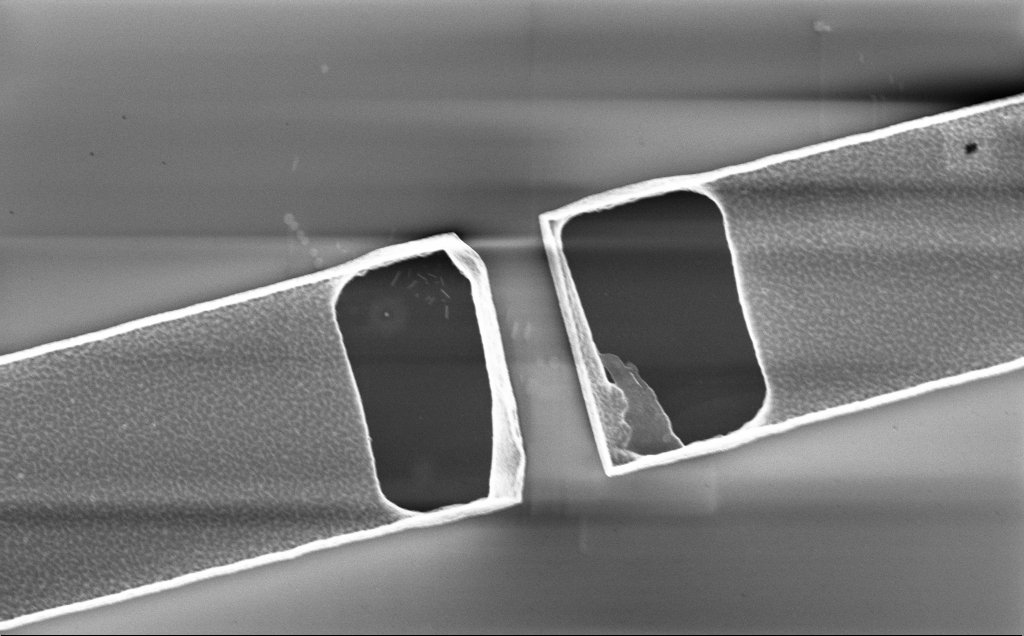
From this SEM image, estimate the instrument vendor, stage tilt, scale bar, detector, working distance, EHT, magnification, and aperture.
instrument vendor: Zeiss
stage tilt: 0°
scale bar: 10000 nm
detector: InLens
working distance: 10 mm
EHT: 2 kV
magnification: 6.16 K X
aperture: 30 µm